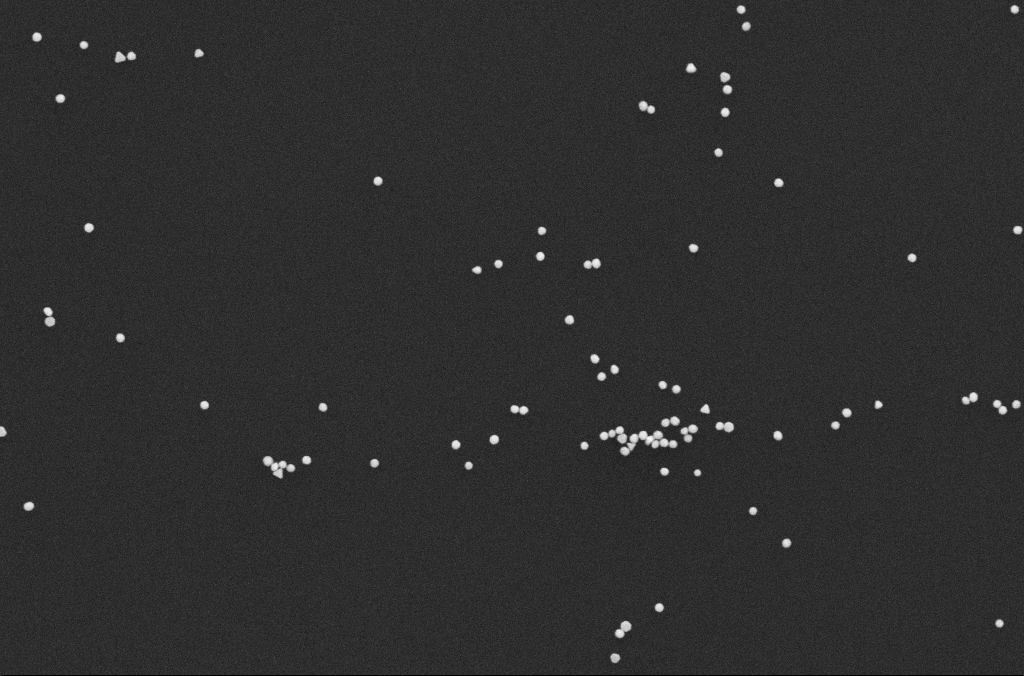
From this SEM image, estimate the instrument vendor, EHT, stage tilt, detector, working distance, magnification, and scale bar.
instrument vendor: Zeiss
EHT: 10 kV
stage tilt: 0°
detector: SE2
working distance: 4.9 mm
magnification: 54.7 K X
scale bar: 1000 nm